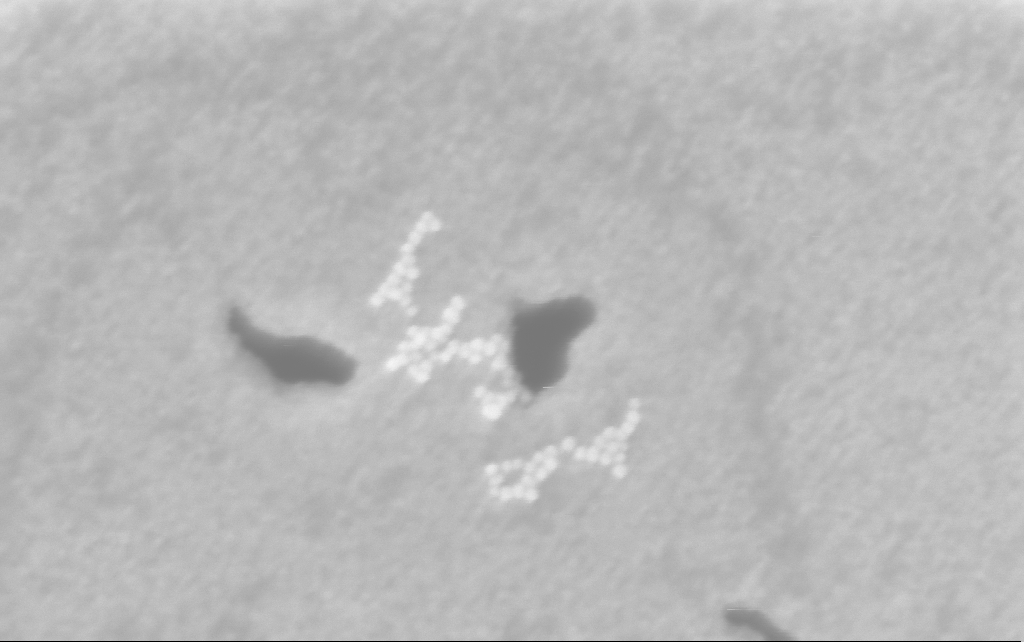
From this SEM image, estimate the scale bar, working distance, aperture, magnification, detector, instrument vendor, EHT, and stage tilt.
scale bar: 200 nm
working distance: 2.5 mm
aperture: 30 µm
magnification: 291.87 K X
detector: InLens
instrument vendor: Zeiss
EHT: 3 kV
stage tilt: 0°